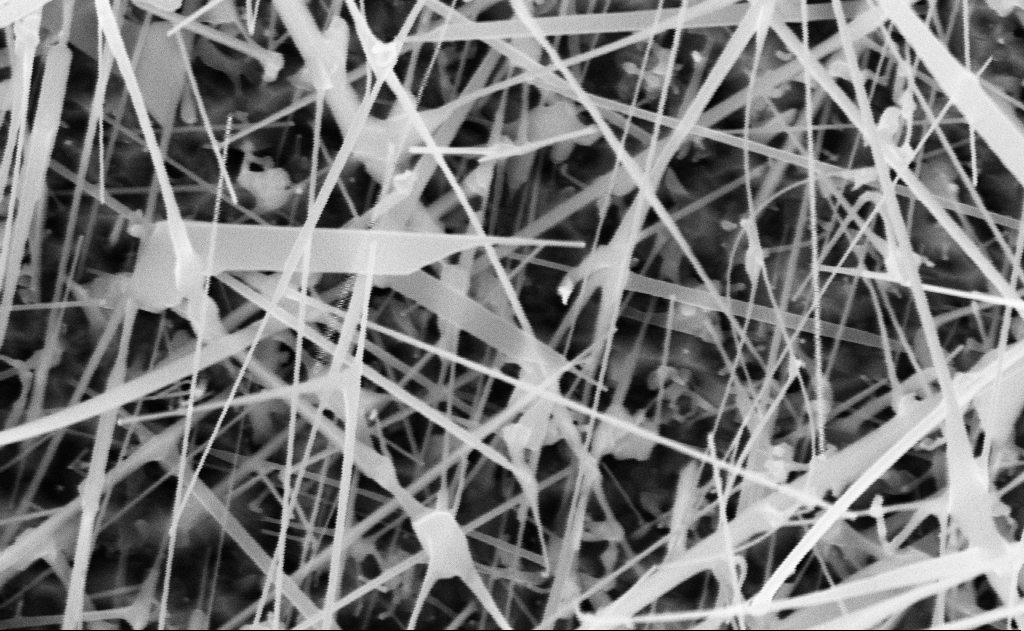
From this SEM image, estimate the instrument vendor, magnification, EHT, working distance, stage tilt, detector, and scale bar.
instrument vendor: Zeiss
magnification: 60 K X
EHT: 10 kV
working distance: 20 mm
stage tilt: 0°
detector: InLens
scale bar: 1000 nm